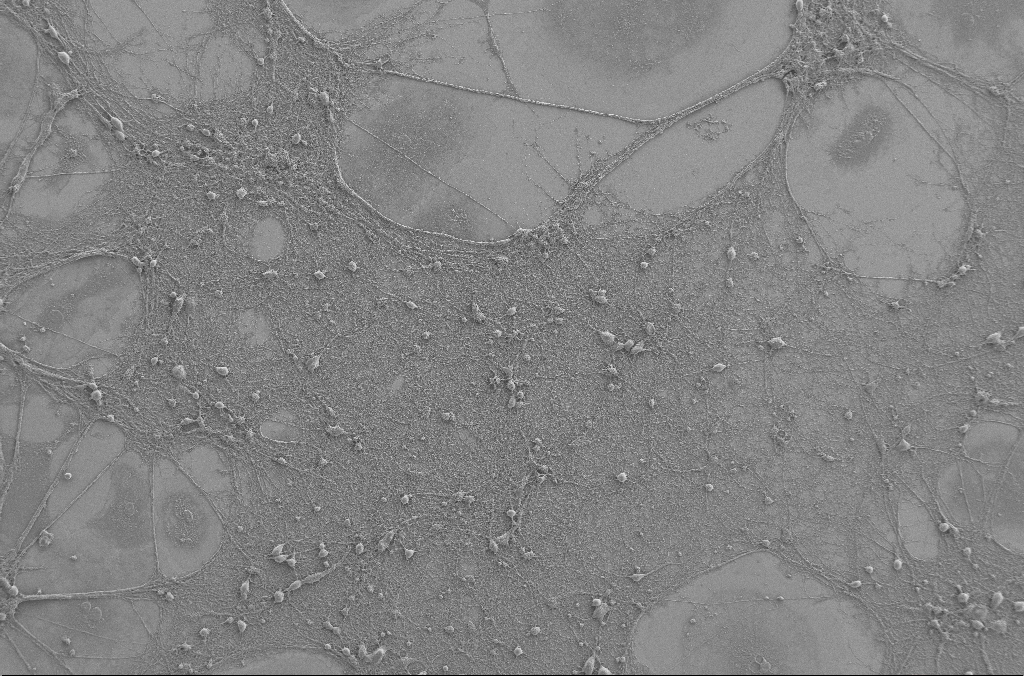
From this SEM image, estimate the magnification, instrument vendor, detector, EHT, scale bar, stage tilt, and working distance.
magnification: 0.5 K X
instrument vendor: Zeiss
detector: SE2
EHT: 2 kV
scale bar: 100000 nm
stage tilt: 0°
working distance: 4 mm